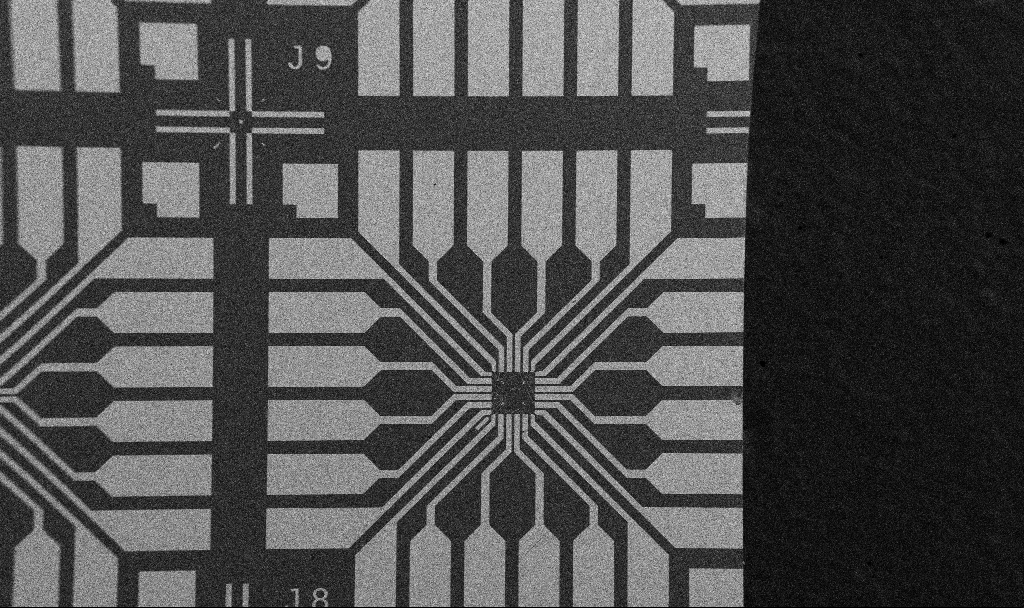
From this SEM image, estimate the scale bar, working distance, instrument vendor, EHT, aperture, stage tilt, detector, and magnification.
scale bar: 200000 nm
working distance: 10.7 mm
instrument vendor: Zeiss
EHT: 5 kV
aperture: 30 µm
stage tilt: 0°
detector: SE2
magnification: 0.1 K X